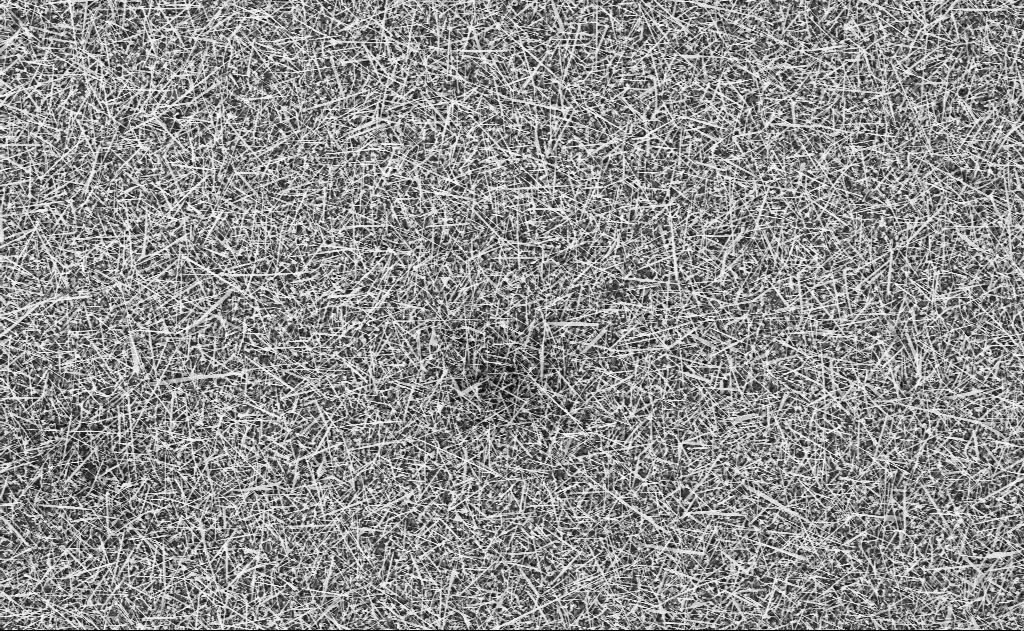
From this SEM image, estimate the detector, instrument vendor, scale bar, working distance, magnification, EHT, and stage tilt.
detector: InLens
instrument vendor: Zeiss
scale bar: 10000 nm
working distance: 20 mm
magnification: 5 K X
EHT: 10 kV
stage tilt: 0°